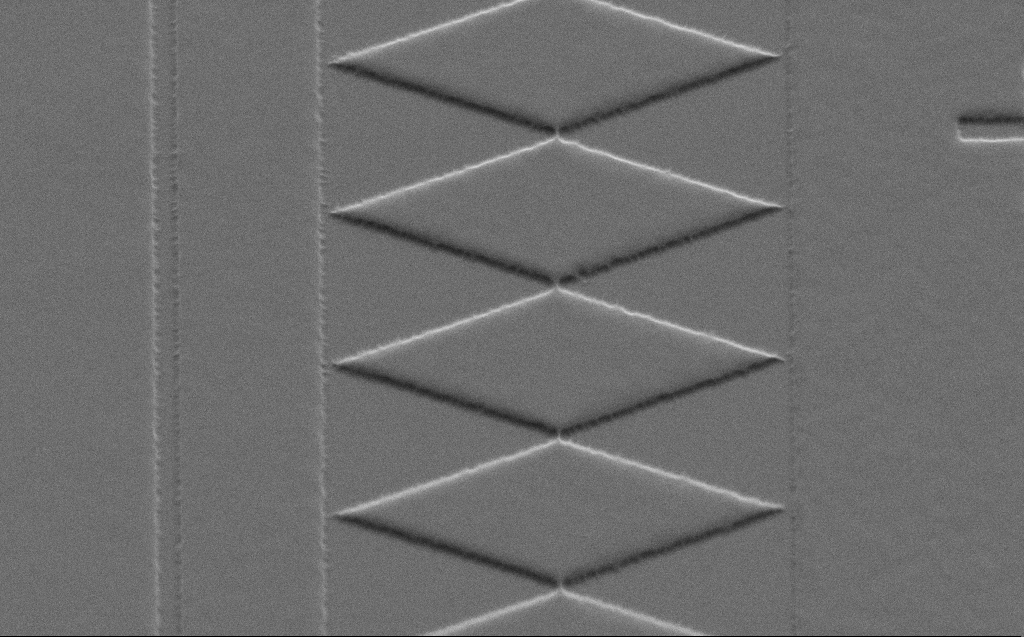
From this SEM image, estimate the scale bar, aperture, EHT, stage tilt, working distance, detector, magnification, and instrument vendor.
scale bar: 10000 nm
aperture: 30 µm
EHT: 3 kV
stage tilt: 45°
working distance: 6 mm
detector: SE2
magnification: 5.69 K X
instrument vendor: Zeiss